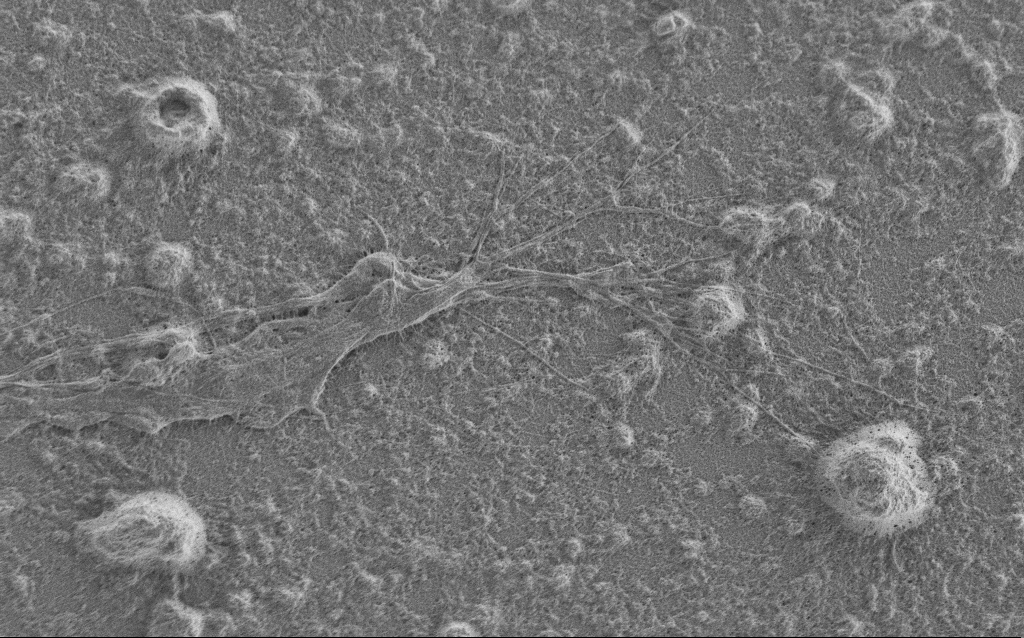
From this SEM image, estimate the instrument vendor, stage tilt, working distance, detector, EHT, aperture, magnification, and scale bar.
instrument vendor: Zeiss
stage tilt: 0°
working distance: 6 mm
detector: SE2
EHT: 1 kV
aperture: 30 µm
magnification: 7.5 K X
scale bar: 2000 nm